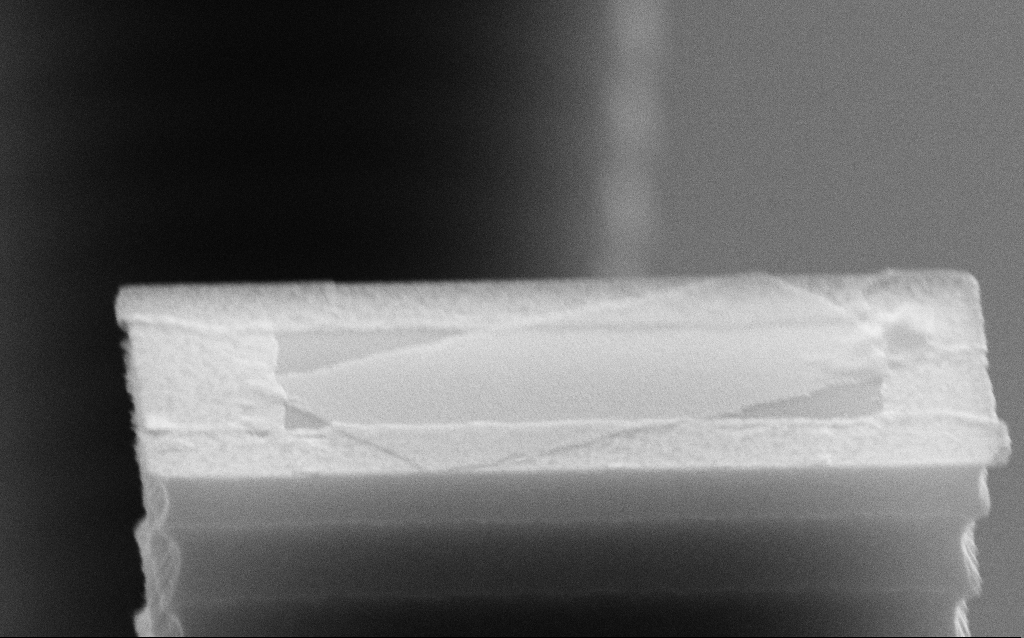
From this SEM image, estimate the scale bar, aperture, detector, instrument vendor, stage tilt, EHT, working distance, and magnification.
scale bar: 200 nm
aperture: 30 µm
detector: SE2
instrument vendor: Zeiss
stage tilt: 70°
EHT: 10 kV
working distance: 7.4 mm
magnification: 106.68 K X